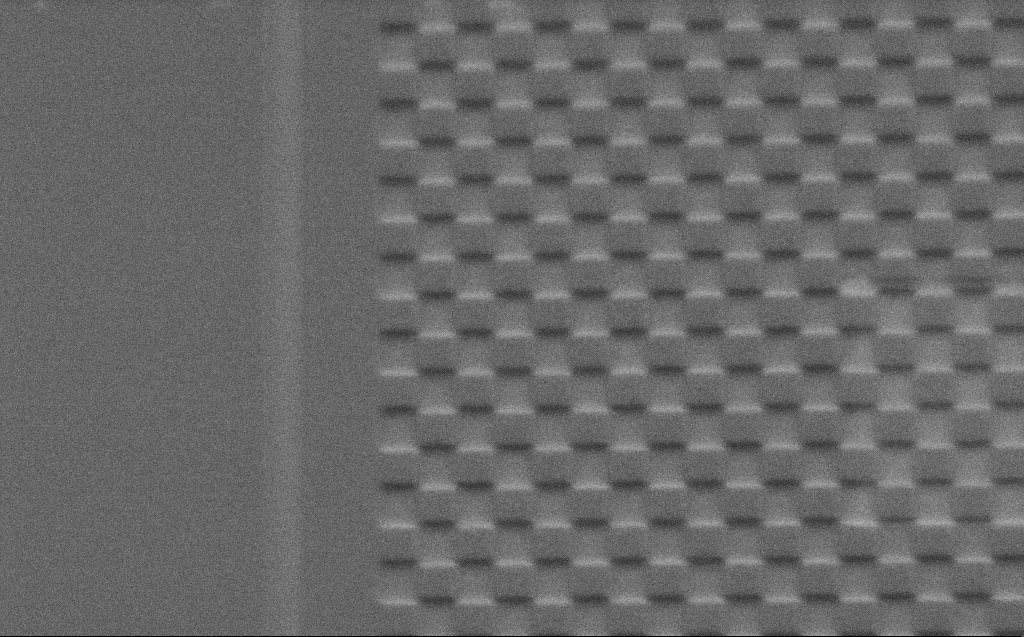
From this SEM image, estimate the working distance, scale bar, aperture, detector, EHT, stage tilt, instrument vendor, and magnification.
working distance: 4 mm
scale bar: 2000 nm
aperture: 30 µm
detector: SE2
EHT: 2 kV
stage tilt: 45°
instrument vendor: Zeiss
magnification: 14.33 K X